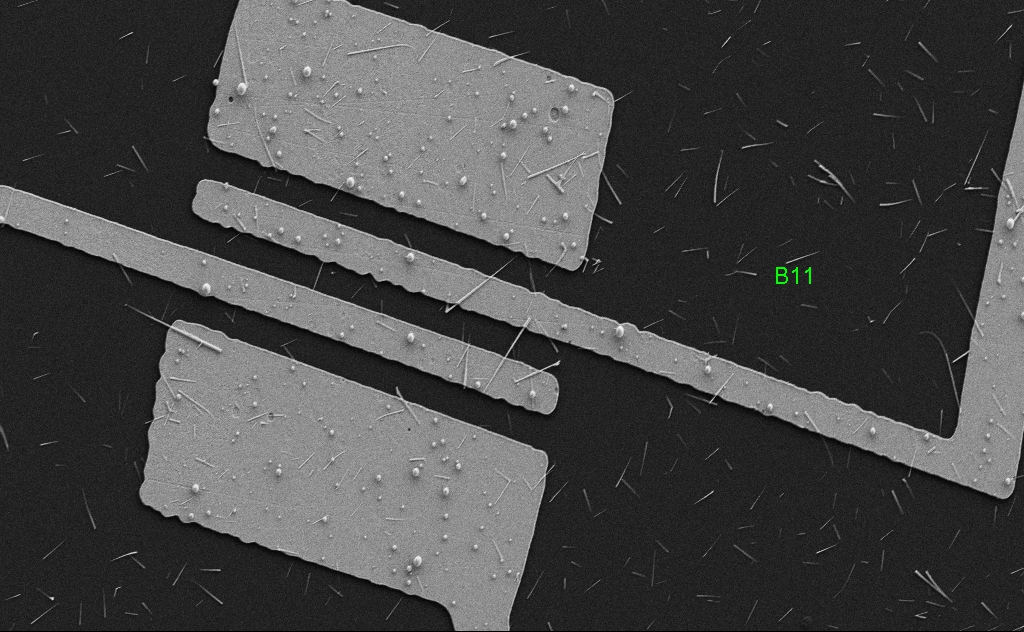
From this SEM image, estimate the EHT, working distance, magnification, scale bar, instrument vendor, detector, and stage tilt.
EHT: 5 kV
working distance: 5 mm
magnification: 5.08 K X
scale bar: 10000 nm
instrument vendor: Zeiss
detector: SE2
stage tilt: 0°